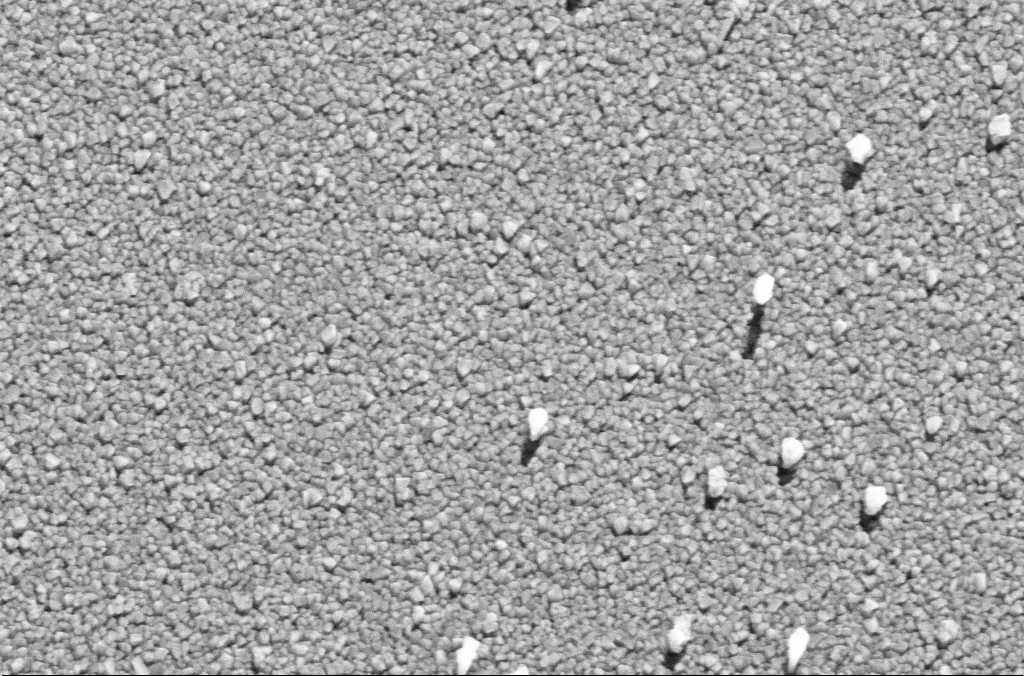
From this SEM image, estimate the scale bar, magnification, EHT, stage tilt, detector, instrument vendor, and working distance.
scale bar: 200 nm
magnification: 131.07 K X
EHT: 20 kV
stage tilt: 0°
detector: SE2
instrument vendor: Zeiss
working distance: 8.5 mm